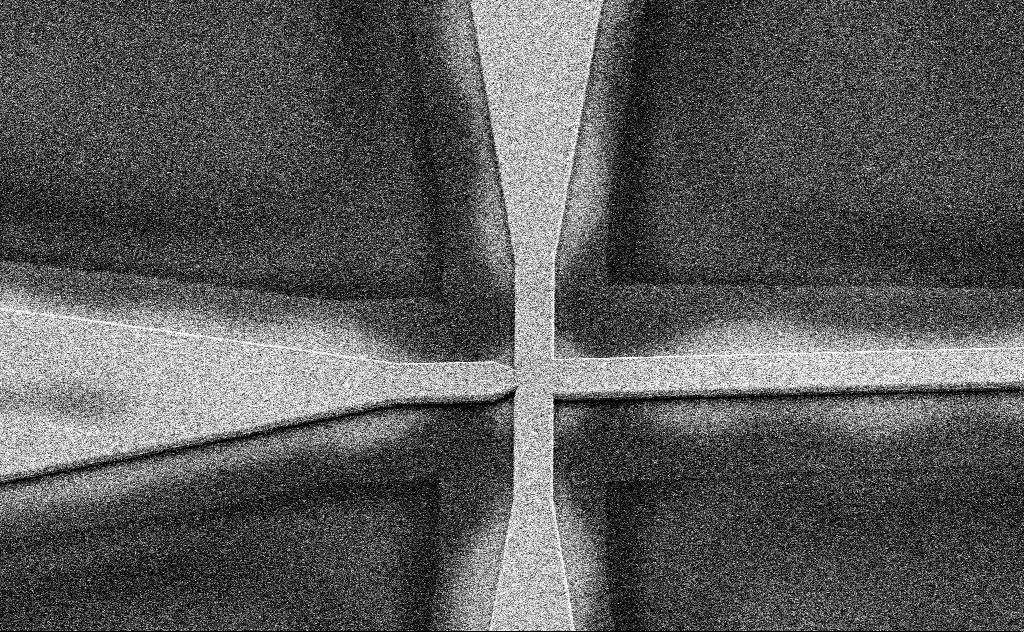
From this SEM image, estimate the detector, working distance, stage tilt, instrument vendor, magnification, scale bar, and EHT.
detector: SE2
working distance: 11 mm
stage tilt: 45°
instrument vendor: Zeiss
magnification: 1.16 K X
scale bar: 20000 nm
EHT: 10 kV